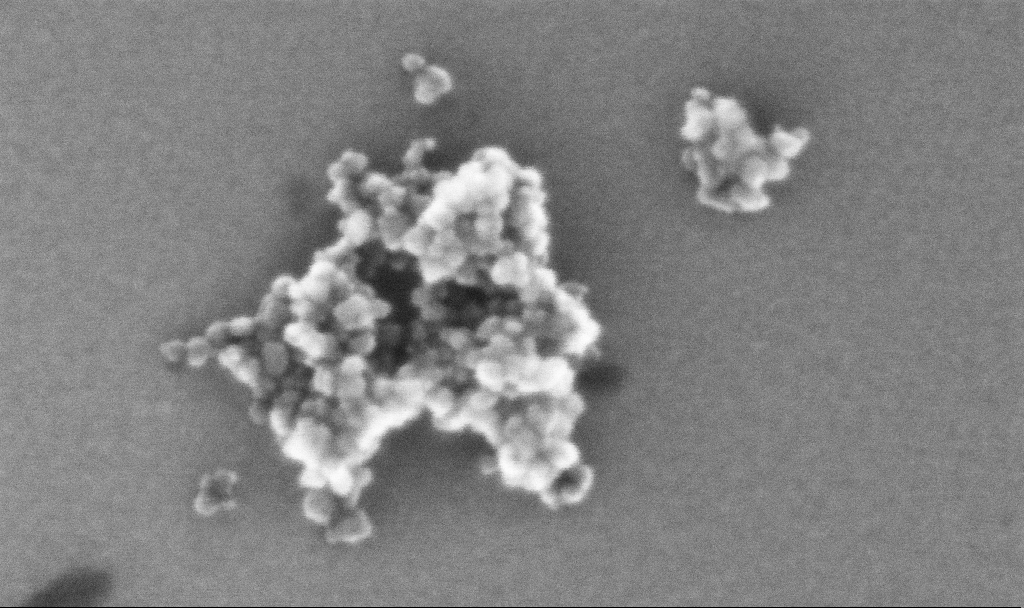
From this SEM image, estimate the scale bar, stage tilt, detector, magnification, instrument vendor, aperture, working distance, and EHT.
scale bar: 100 nm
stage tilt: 0°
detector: InLens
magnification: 616.76 K X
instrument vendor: Zeiss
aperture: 30 µm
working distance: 5.4 mm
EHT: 10 kV